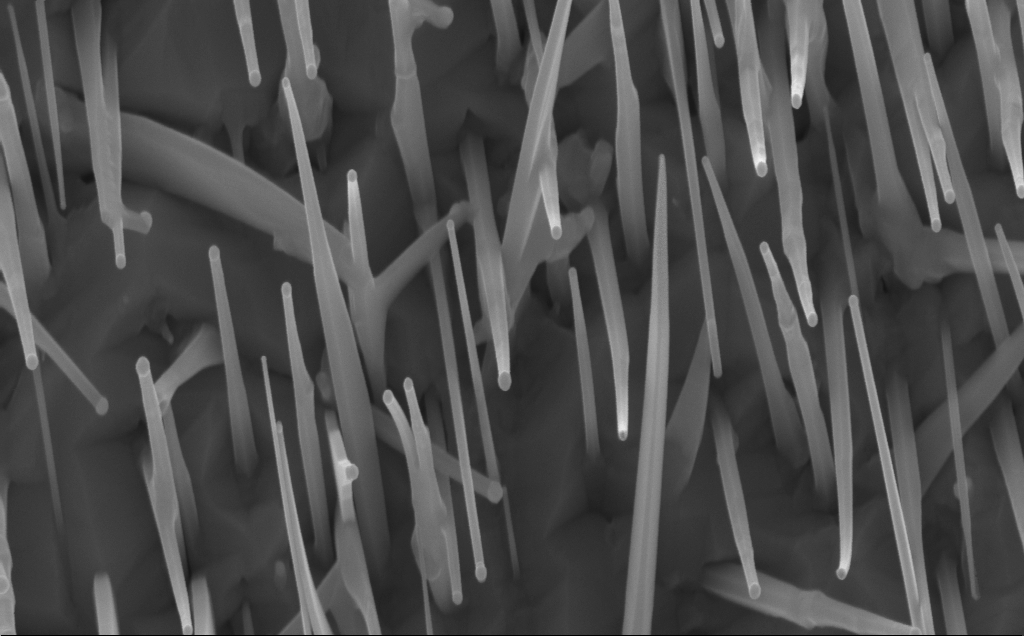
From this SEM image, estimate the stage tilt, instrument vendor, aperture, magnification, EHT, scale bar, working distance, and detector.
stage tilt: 0°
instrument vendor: Zeiss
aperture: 30 µm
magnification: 80 K X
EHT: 10 kV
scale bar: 200 nm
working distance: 7 mm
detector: InLens